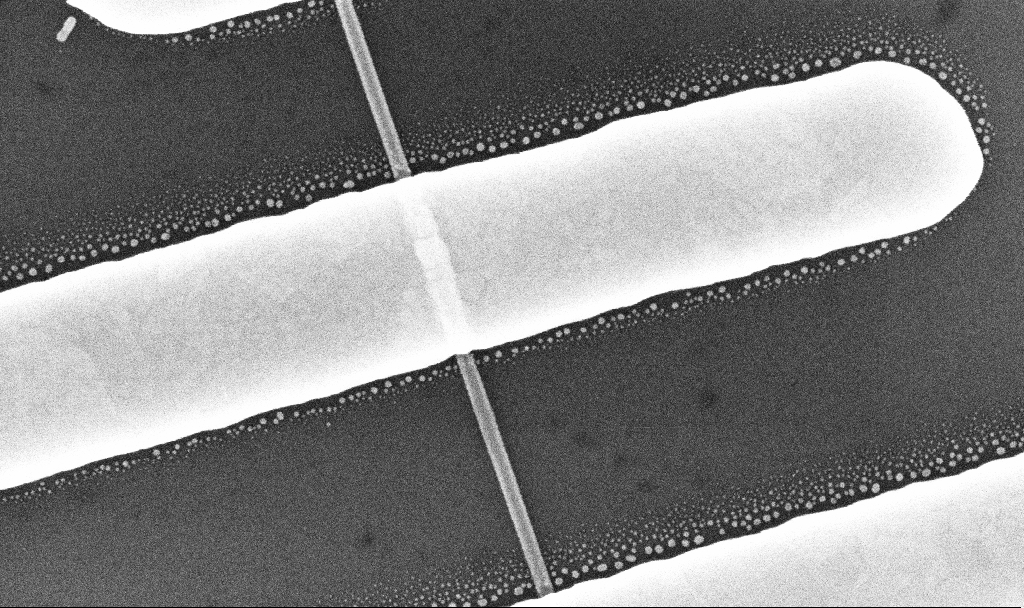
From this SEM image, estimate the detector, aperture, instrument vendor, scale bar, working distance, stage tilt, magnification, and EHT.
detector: InLens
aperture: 30 µm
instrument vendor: Zeiss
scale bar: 200 nm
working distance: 7 mm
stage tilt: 0°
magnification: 156.83 K X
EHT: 10 kV